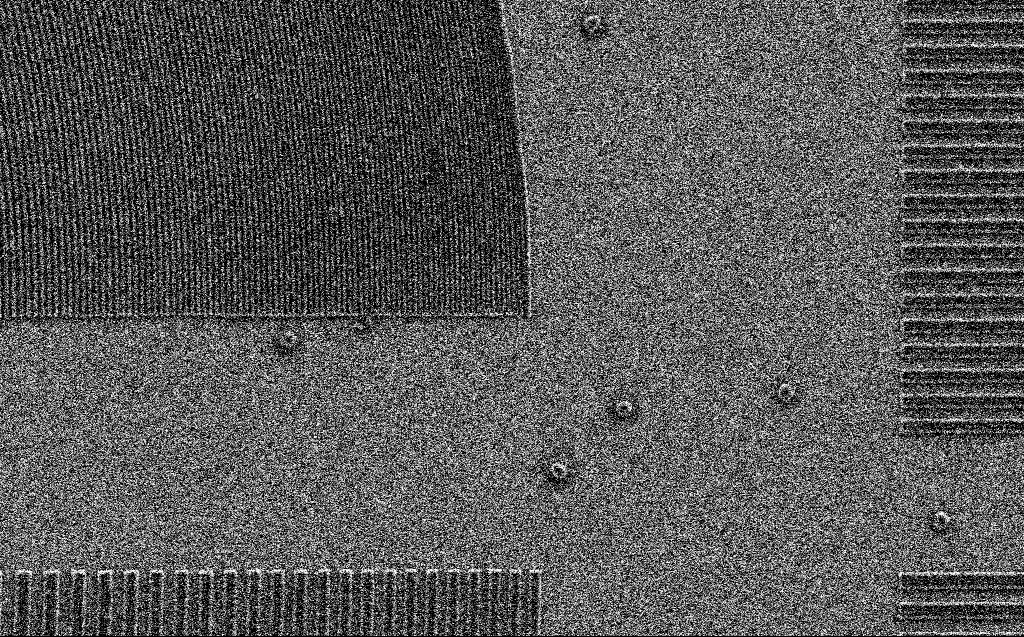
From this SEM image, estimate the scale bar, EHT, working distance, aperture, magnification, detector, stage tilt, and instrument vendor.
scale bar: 2000 nm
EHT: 3 kV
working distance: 6 mm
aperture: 30 µm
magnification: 9.29 K X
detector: SE2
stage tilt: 0°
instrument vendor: Zeiss